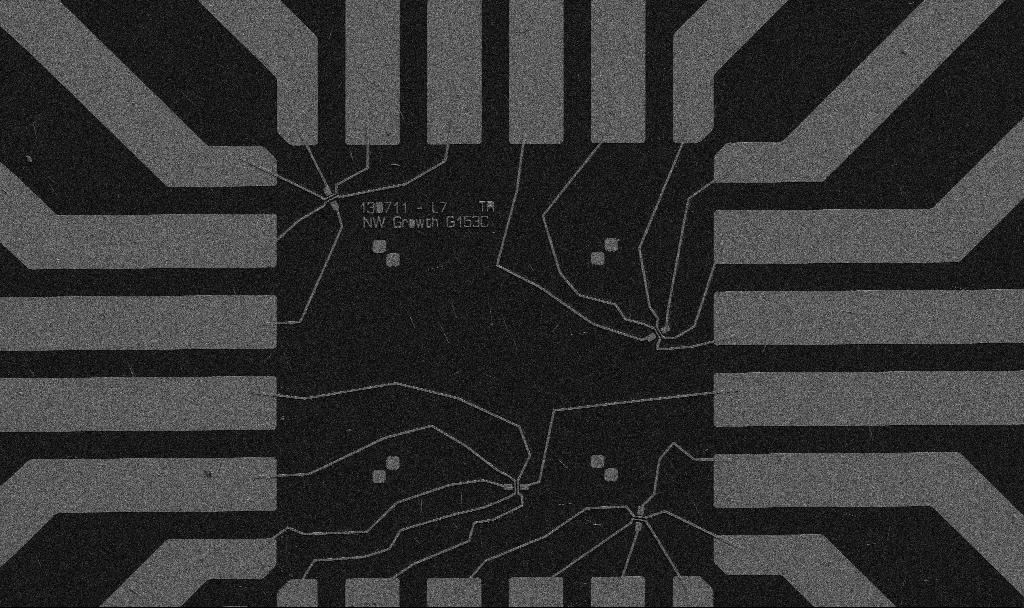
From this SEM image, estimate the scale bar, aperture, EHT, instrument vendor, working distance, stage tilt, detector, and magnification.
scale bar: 20000 nm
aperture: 30 µm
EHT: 5 kV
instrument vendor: Zeiss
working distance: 10.7 mm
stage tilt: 0°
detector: SE2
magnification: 1 K X